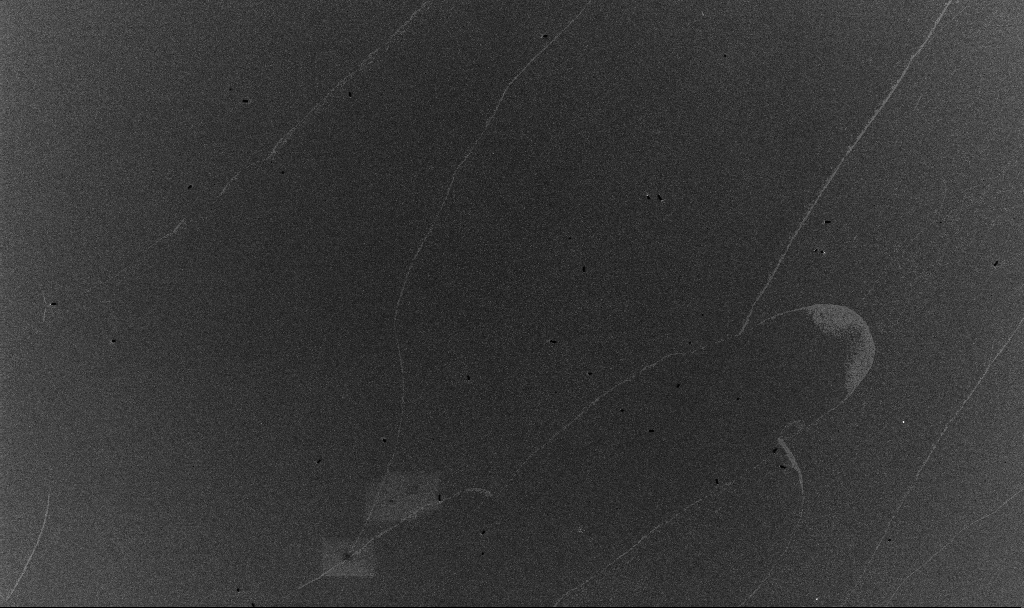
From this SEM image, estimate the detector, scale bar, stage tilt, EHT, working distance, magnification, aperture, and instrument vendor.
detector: InLens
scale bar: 20000 nm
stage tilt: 0°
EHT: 10 kV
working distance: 3.4 mm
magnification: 1 K X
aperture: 30 µm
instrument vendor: Zeiss